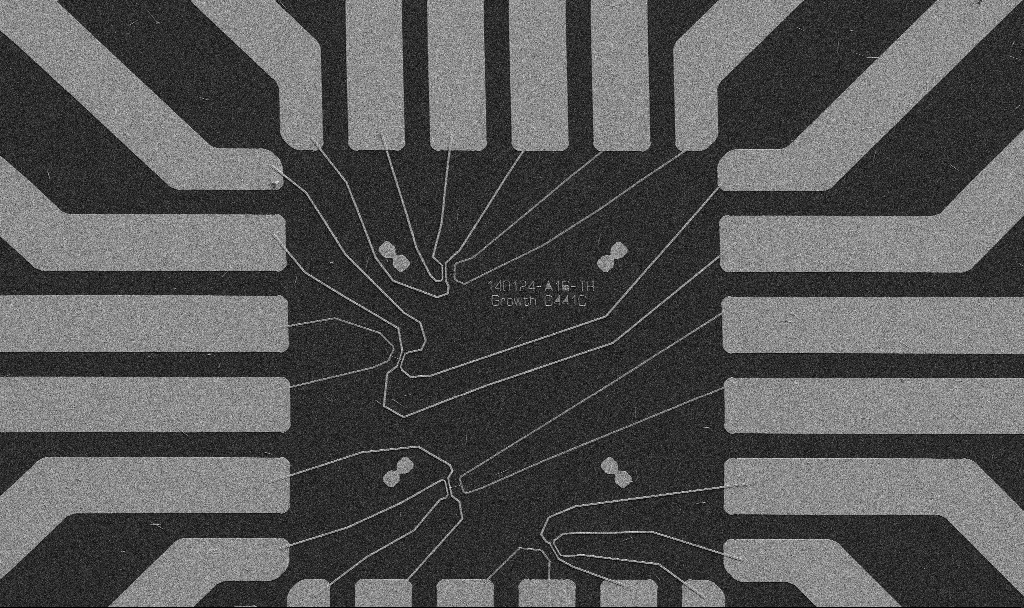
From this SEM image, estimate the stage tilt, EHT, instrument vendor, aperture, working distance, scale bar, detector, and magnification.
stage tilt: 0°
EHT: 5 kV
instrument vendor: Zeiss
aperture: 30 µm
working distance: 10.7 mm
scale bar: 20000 nm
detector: SE2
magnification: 1 K X